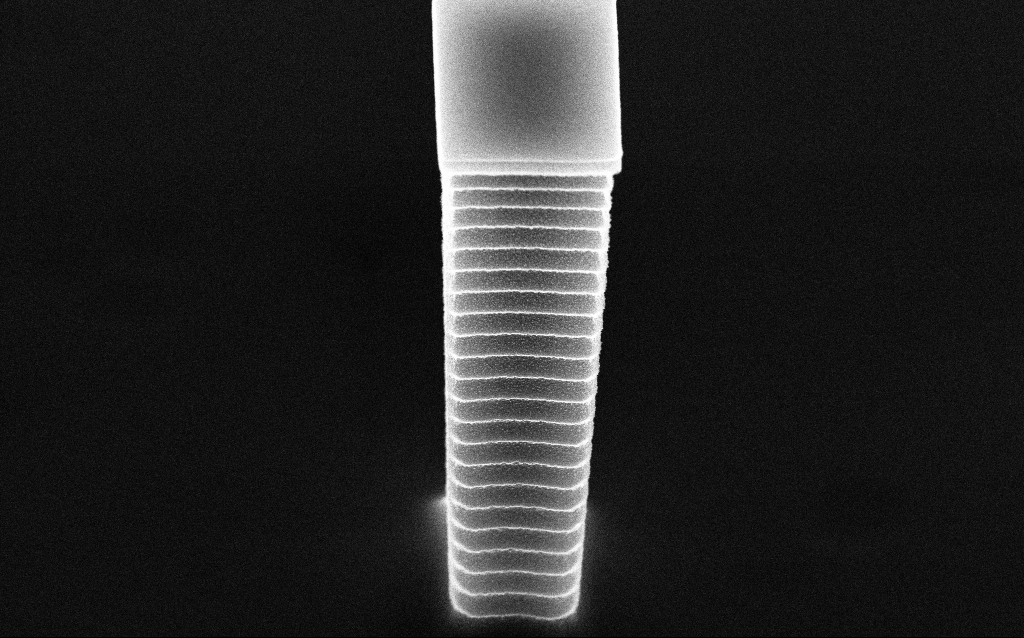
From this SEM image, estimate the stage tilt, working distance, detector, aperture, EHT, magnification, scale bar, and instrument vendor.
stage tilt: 45°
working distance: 4.8 mm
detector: InLens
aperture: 30 µm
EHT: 10 kV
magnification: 33.26 K X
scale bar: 1000 nm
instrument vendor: Zeiss